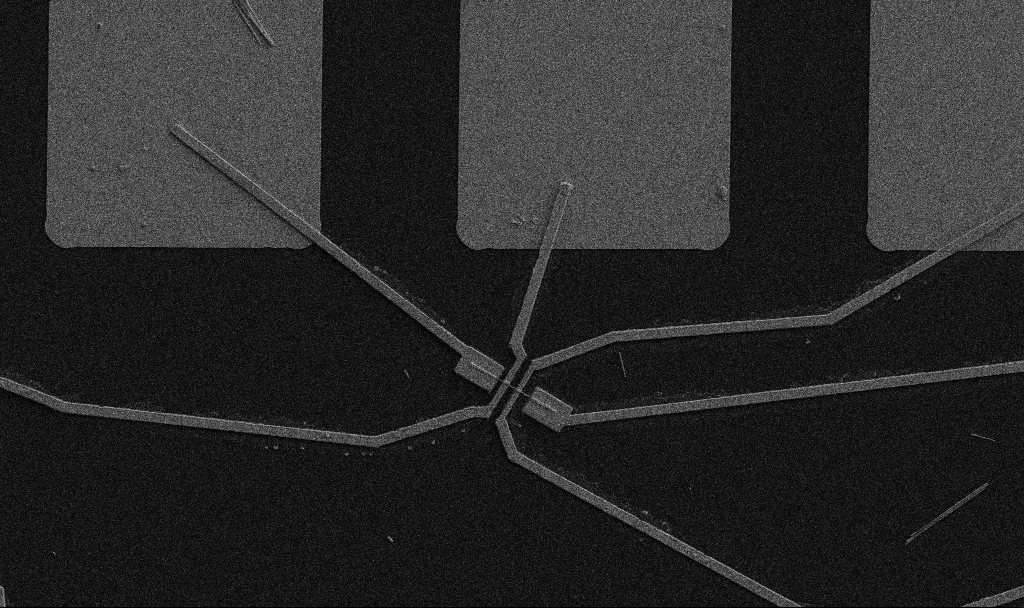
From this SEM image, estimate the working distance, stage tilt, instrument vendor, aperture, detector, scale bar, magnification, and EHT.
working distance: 10.7 mm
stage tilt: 0°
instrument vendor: Zeiss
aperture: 30 µm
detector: SE2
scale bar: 10000 nm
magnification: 5 K X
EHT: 5 kV